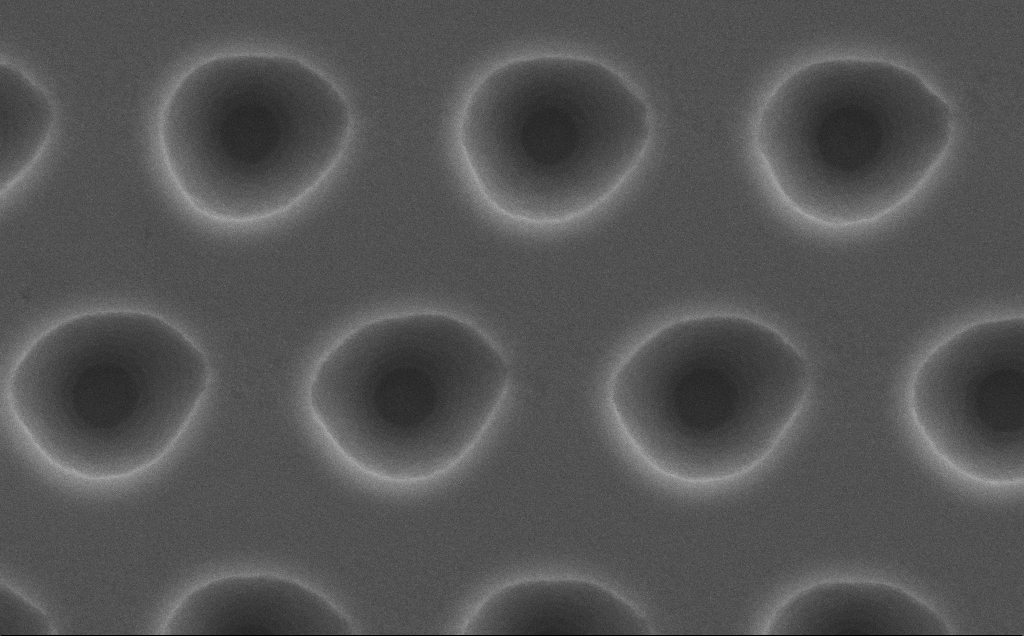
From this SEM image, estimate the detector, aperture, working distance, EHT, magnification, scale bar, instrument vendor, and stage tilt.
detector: SE2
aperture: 30 µm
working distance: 8 mm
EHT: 5 kV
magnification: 27.35 K X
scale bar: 2000 nm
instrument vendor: Zeiss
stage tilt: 0°